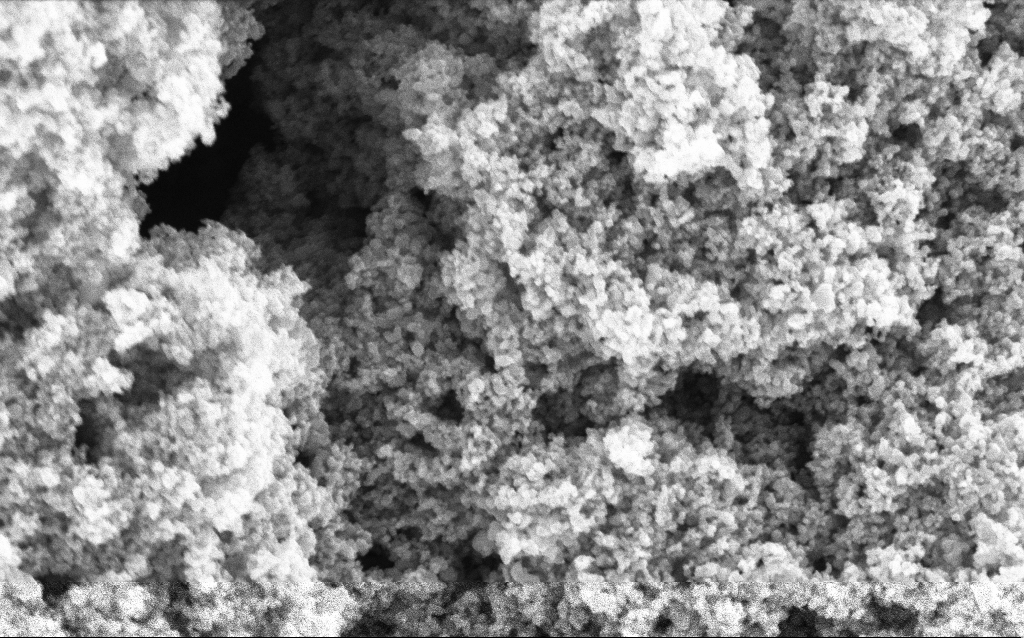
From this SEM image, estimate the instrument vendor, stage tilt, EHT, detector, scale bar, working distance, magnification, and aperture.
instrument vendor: Zeiss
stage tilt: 0°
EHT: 5 kV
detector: InLens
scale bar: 200 nm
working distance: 4.6 mm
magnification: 88.07 K X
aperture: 30 µm